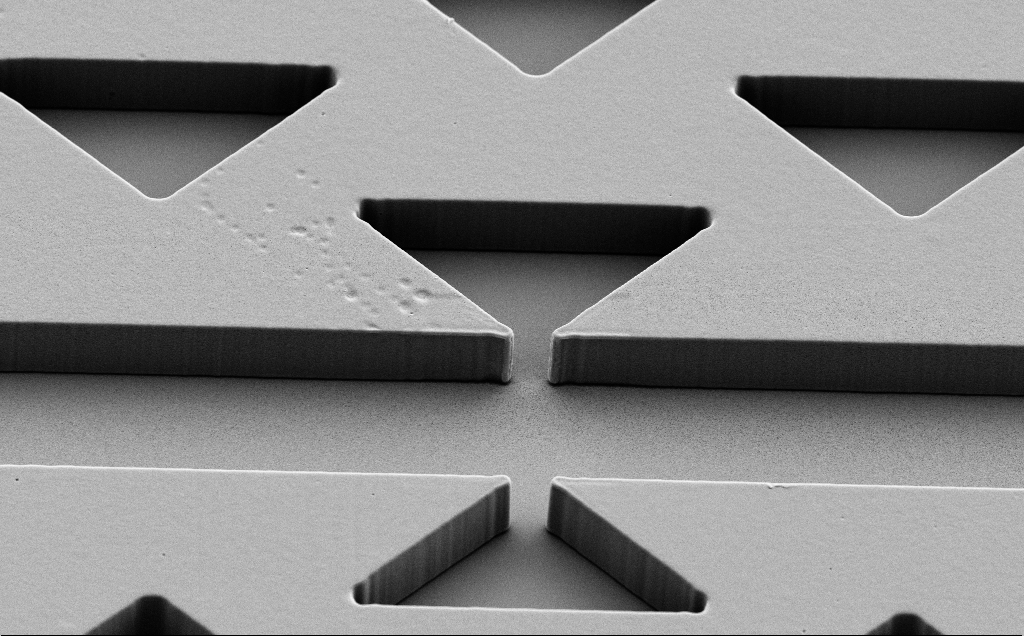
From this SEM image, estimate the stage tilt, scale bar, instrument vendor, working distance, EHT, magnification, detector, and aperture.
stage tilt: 40°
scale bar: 10000 nm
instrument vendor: Zeiss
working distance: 8 mm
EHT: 5 kV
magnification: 3.56 K X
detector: SE2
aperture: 30 µm